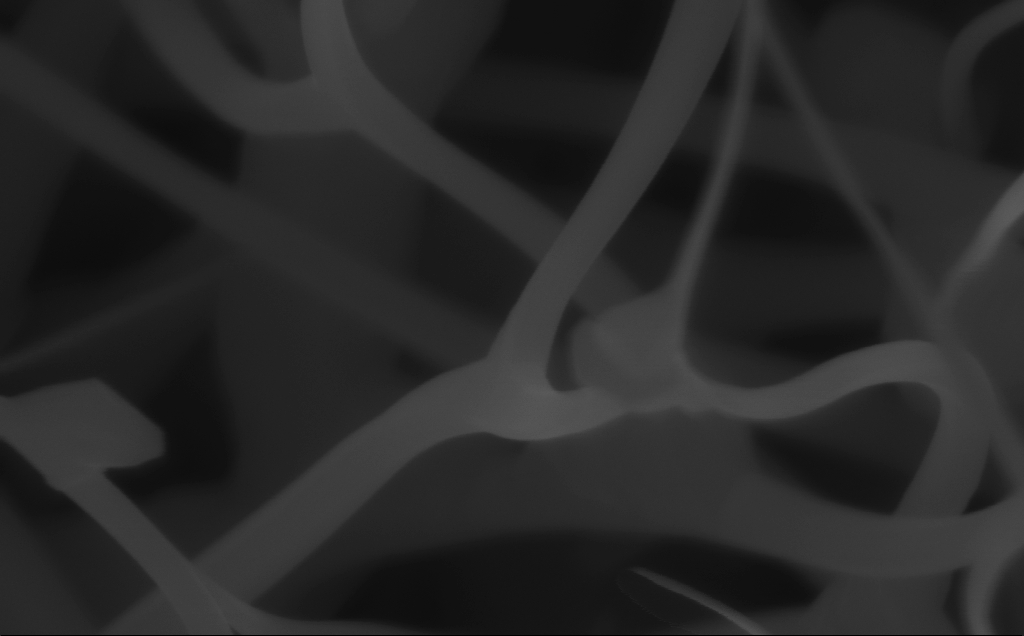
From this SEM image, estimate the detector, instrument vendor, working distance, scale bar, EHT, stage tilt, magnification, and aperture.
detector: InLens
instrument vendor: Zeiss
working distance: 6 mm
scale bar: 200 nm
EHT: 10 kV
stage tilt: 0°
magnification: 235.88 K X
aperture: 30 µm